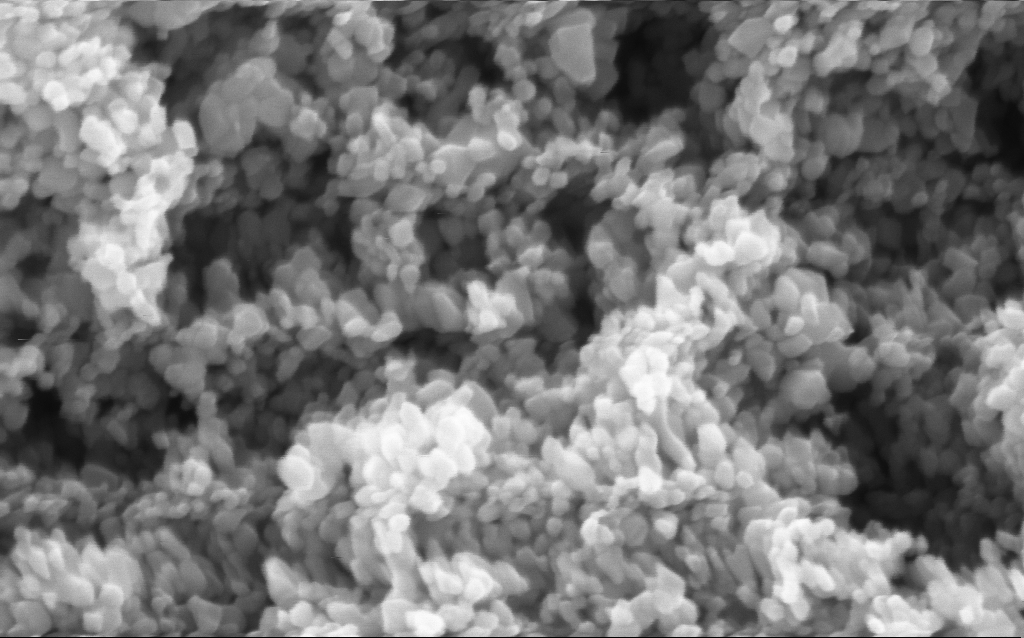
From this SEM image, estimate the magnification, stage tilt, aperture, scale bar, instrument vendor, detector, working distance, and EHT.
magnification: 294.55 K X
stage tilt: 0°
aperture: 30 µm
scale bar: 200 nm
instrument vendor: Zeiss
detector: InLens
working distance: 4.7 mm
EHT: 5 kV